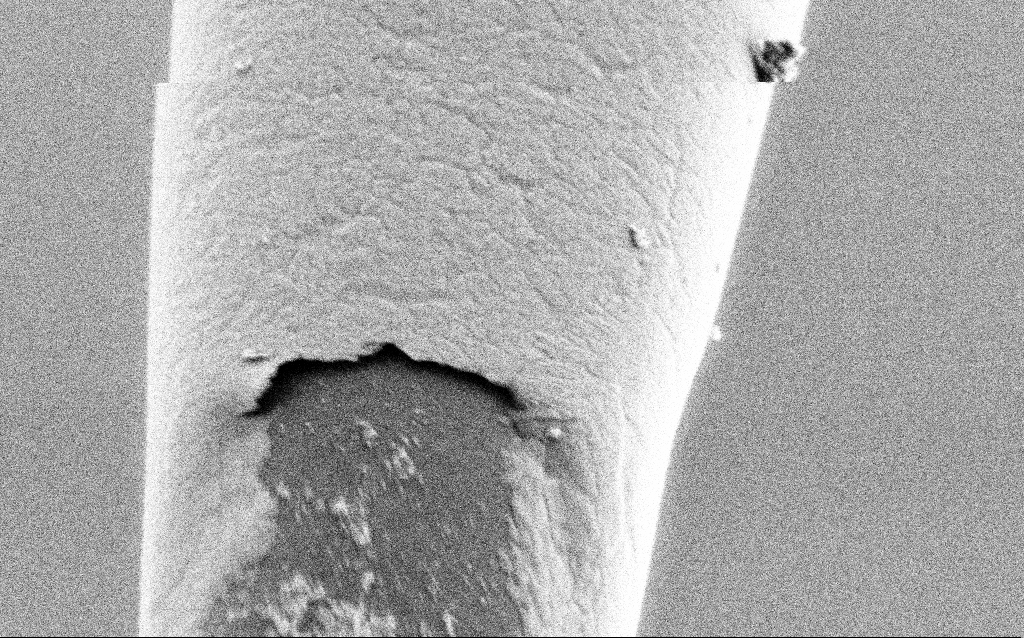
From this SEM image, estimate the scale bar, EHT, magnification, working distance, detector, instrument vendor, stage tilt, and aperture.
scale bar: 200 nm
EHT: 1 kV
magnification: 75 K X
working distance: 6.8 mm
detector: SE2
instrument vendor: Zeiss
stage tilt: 45°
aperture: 30 µm